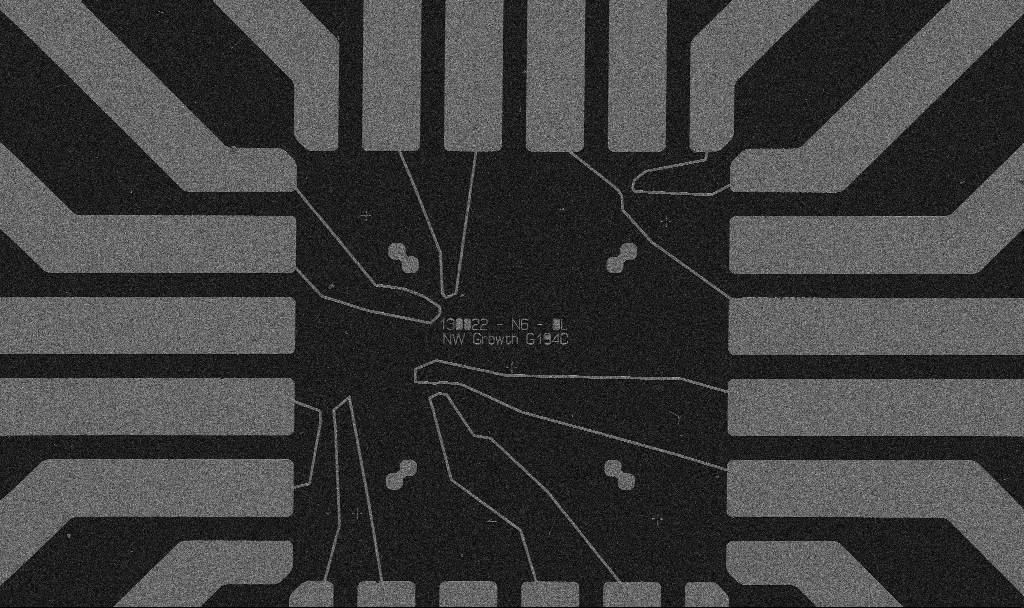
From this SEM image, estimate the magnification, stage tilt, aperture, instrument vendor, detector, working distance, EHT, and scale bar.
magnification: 1 K X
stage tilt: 0°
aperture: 30 µm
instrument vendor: Zeiss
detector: SE2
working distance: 10.7 mm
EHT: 5 kV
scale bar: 20000 nm